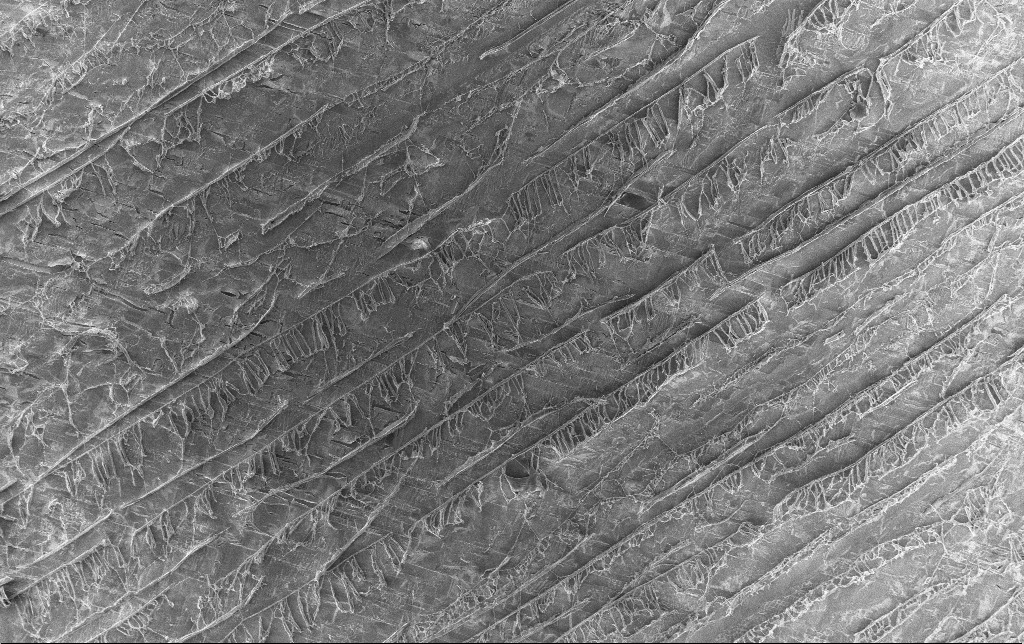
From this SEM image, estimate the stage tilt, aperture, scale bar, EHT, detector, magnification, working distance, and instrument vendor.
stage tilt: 0°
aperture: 30 µm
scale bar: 20000 nm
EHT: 1.5 kV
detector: InLens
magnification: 0.812 K X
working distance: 3.1 mm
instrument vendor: Zeiss